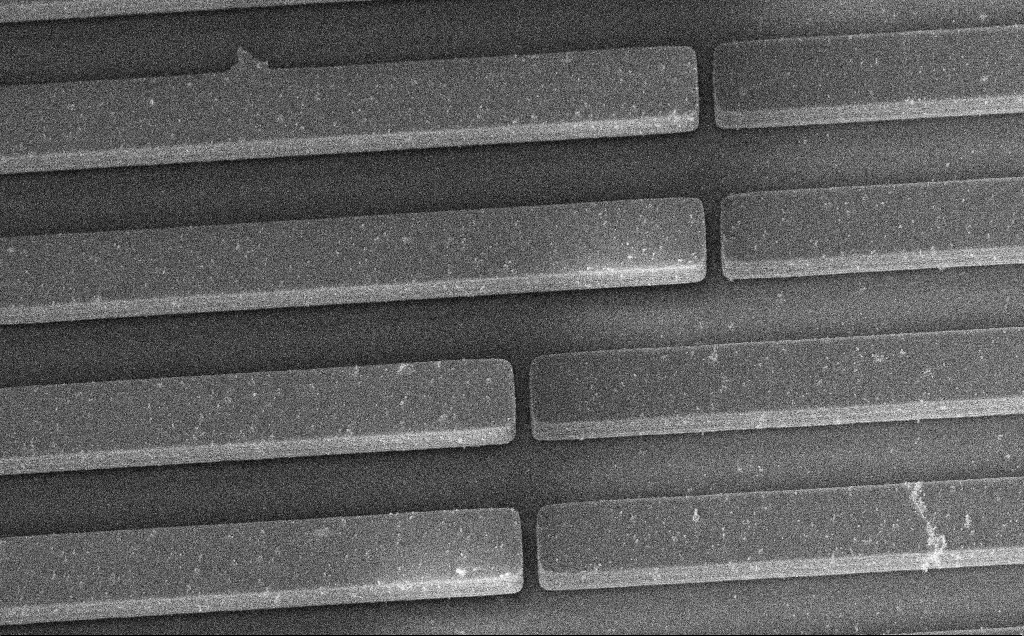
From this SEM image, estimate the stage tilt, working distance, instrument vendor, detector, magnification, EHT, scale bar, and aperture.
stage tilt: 45°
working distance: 10 mm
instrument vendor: Zeiss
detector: InLens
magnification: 2.91 K X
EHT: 7.5 kV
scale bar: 10000 nm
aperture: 30 µm